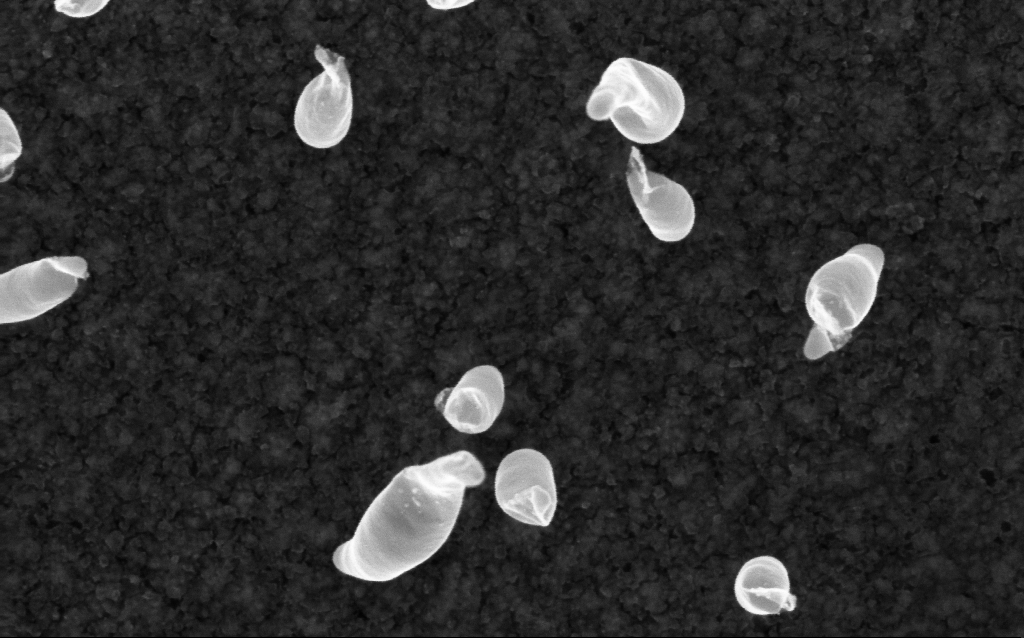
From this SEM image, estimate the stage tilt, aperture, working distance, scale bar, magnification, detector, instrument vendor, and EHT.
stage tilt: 0°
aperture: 30 µm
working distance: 2.1 mm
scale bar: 100 nm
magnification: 200 K X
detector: InLens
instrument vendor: Zeiss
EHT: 5 kV